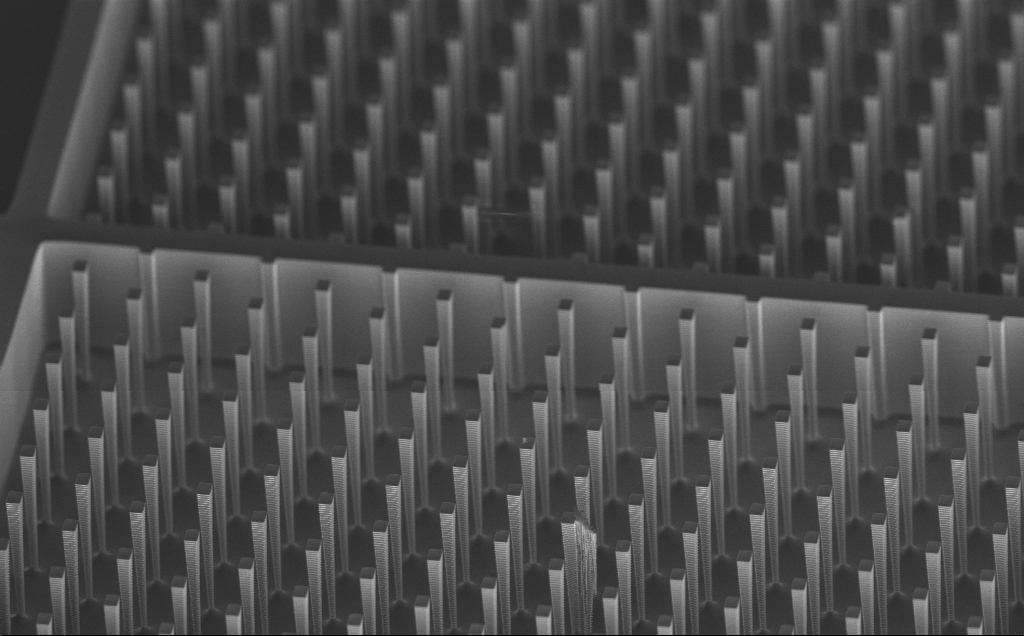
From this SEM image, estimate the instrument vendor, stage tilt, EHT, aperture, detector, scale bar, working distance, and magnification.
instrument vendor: Zeiss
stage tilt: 60°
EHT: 3 kV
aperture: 30 µm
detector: InLens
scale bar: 20000 nm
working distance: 5.2 mm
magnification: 2.49 K X